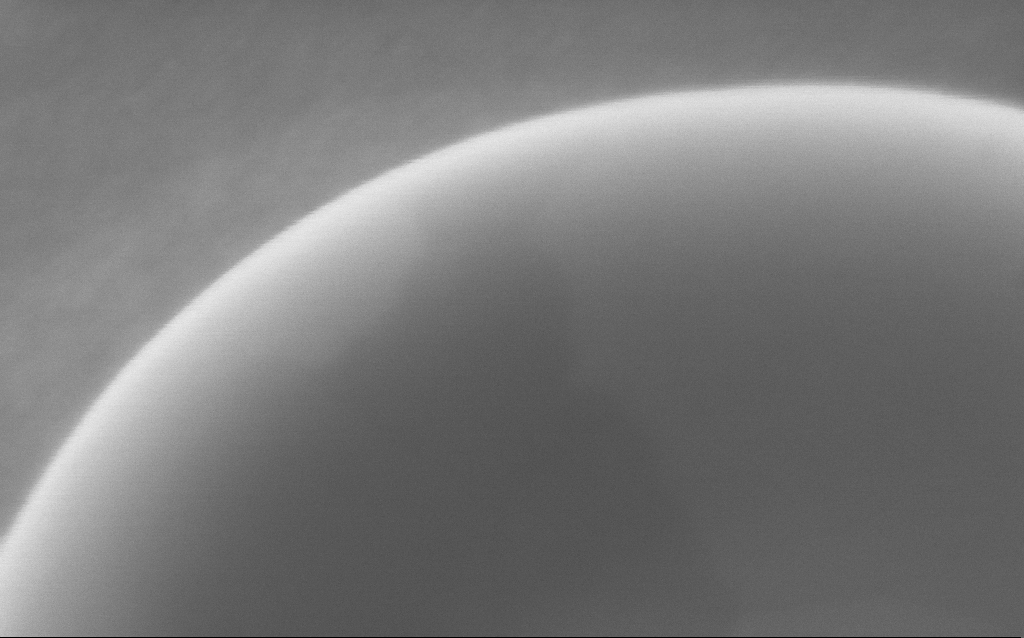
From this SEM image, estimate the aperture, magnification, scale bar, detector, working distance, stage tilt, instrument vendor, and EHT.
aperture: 30 µm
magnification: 404.29 K X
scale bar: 100 nm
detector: InLens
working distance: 3 mm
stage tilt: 0°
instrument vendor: Zeiss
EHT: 5 kV